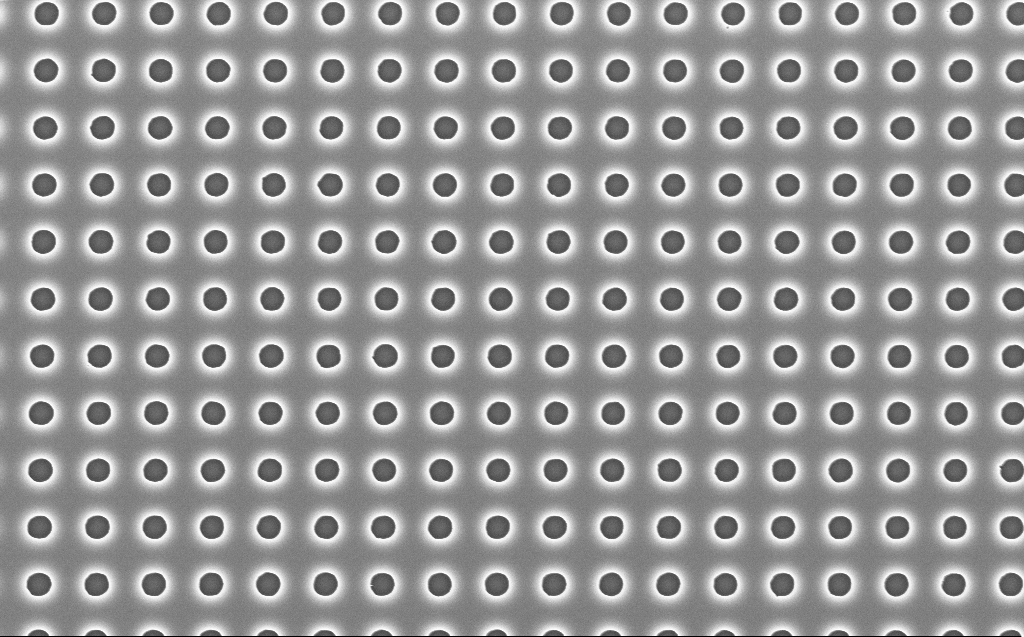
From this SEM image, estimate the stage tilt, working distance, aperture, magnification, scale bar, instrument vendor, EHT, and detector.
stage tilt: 0°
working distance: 7 mm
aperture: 30 µm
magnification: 20 K X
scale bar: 2000 nm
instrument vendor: Zeiss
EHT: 5 kV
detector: InLens